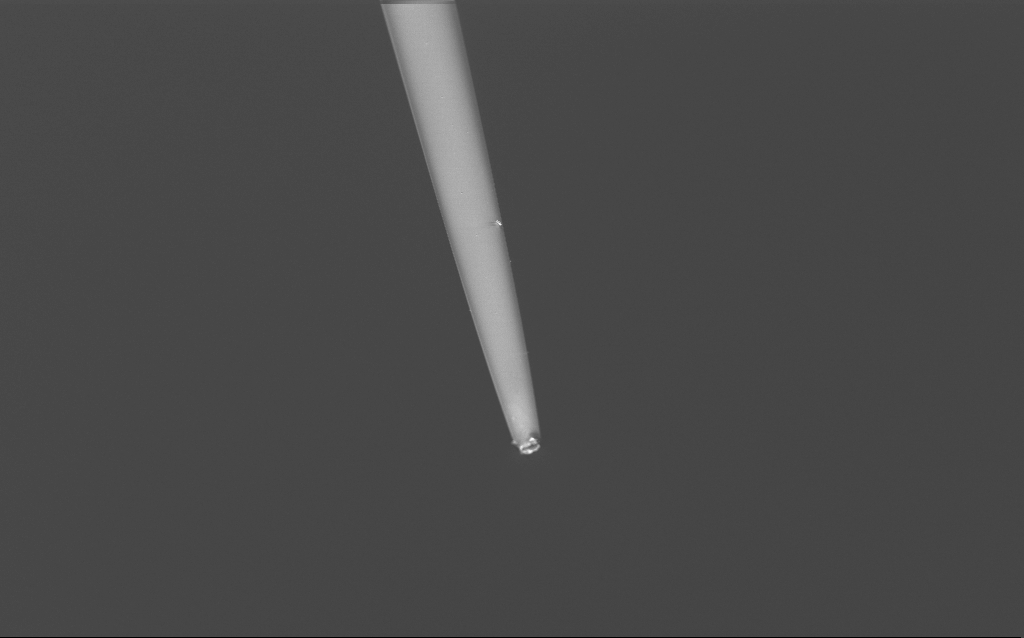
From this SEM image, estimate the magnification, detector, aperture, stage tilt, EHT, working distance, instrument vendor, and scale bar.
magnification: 1 K X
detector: InLens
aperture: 30 µm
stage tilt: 45°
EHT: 2 kV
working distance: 6 mm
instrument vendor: Zeiss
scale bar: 20000 nm